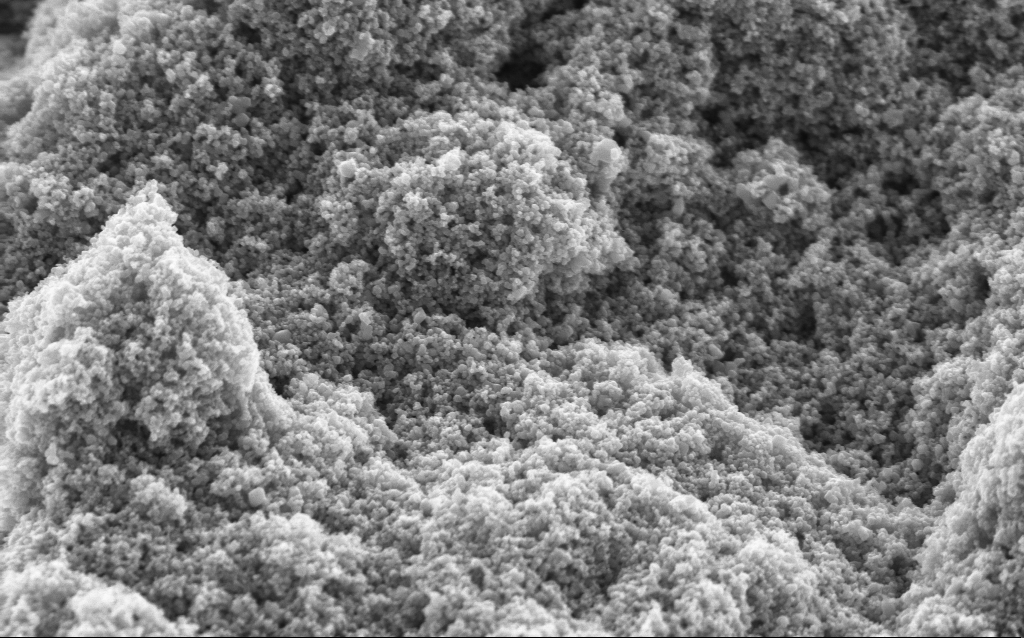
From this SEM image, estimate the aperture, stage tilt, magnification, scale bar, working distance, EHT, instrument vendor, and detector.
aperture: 30 µm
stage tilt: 0°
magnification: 68.59 K X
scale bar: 1000 nm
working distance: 4 mm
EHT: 5 kV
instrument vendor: Zeiss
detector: InLens